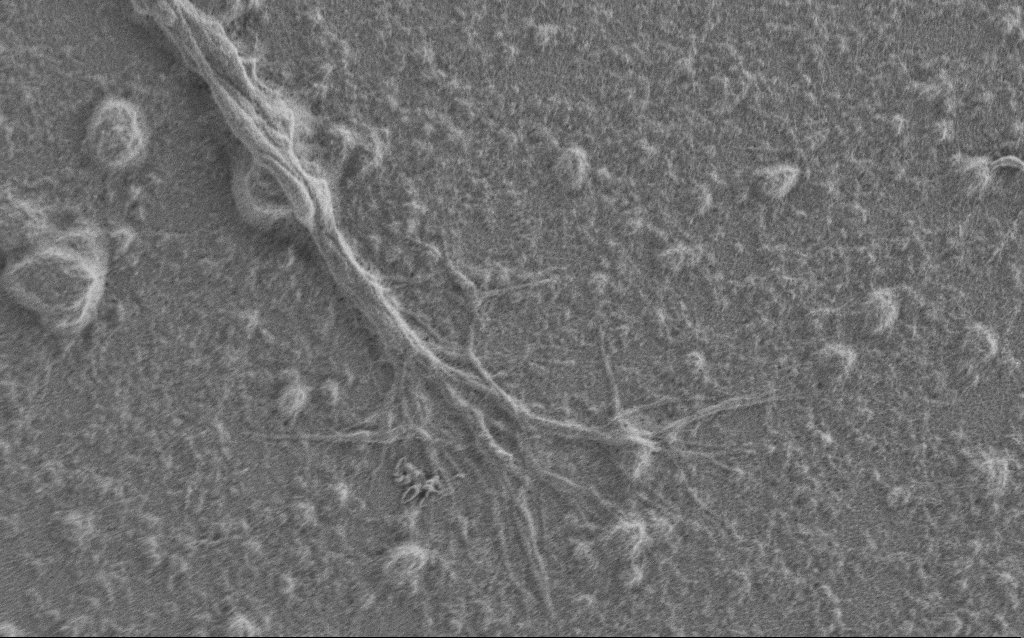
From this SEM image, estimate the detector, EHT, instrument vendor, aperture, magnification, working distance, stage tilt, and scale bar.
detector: SE2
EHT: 1 kV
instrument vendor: Zeiss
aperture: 30 µm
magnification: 7.5 K X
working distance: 6 mm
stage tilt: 0°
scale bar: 2000 nm